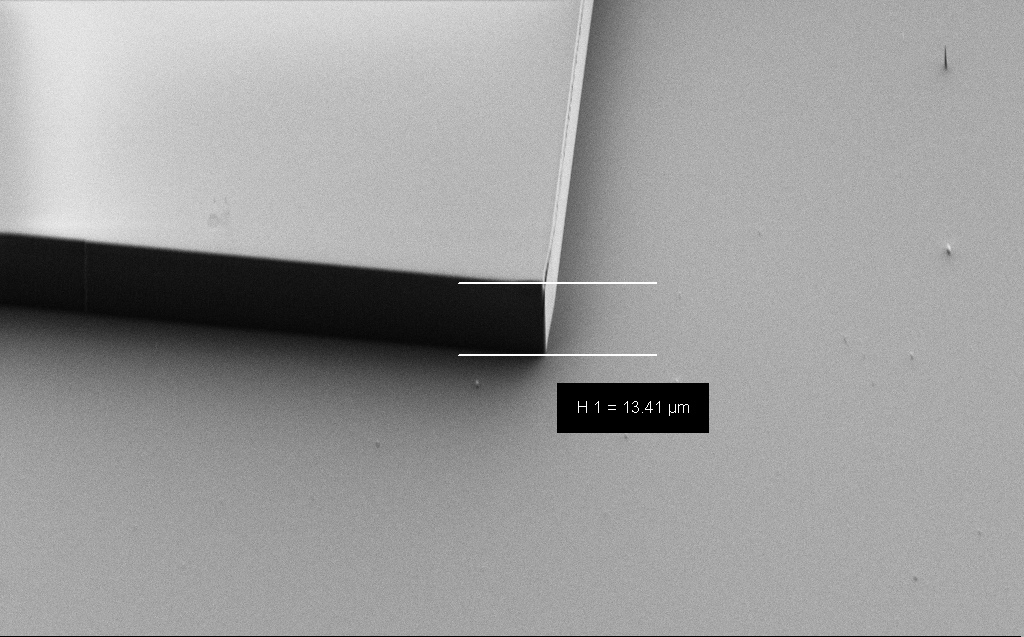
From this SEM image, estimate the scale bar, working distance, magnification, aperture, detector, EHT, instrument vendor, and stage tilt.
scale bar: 20000 nm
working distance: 7 mm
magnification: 1.97 K X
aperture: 30 µm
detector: SE2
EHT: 1.1 kV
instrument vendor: Zeiss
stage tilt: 45°